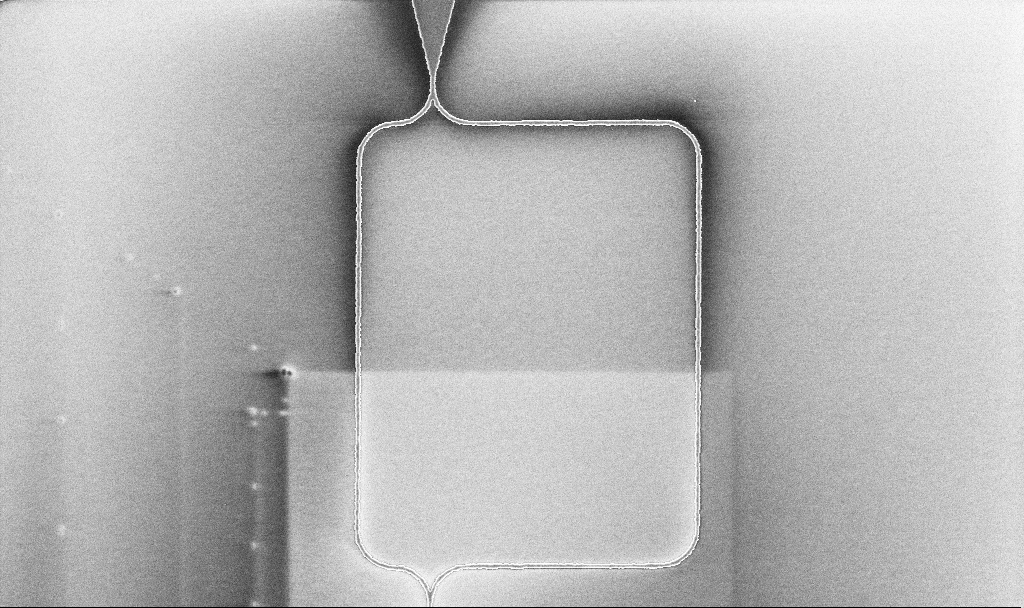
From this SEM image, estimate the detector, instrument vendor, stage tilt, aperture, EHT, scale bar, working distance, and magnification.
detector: InLens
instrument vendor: Zeiss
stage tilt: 0°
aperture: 30 µm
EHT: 5 kV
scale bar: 10000 nm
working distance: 10.1 mm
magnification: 2.86 K X